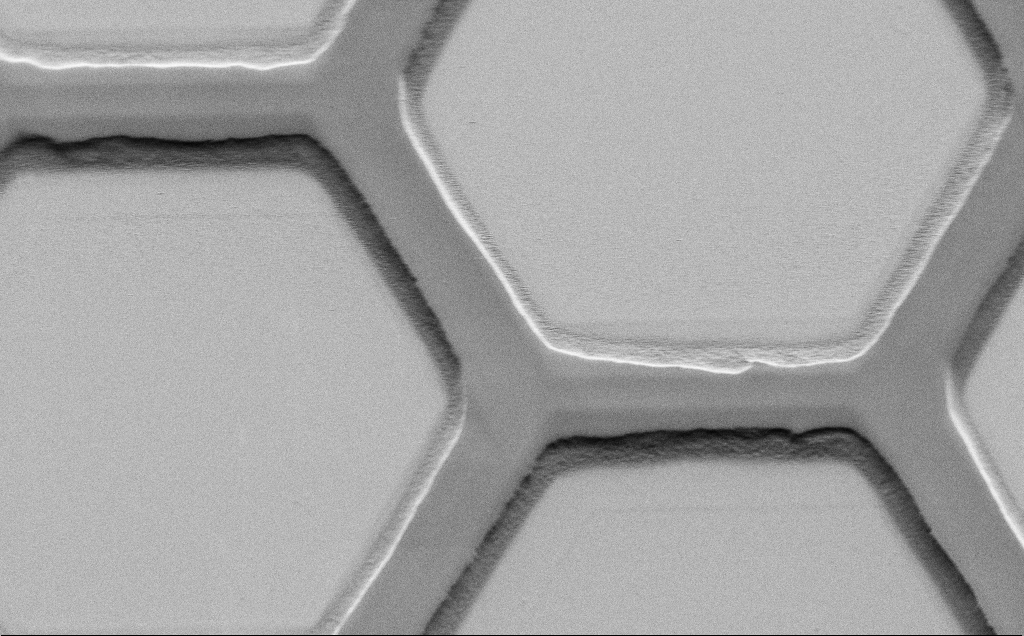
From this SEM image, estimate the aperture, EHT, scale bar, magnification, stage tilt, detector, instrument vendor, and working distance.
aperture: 30 µm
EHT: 1.5 kV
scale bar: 10000 nm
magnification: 3.81 K X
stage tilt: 45°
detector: SE2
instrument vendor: Zeiss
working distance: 7 mm